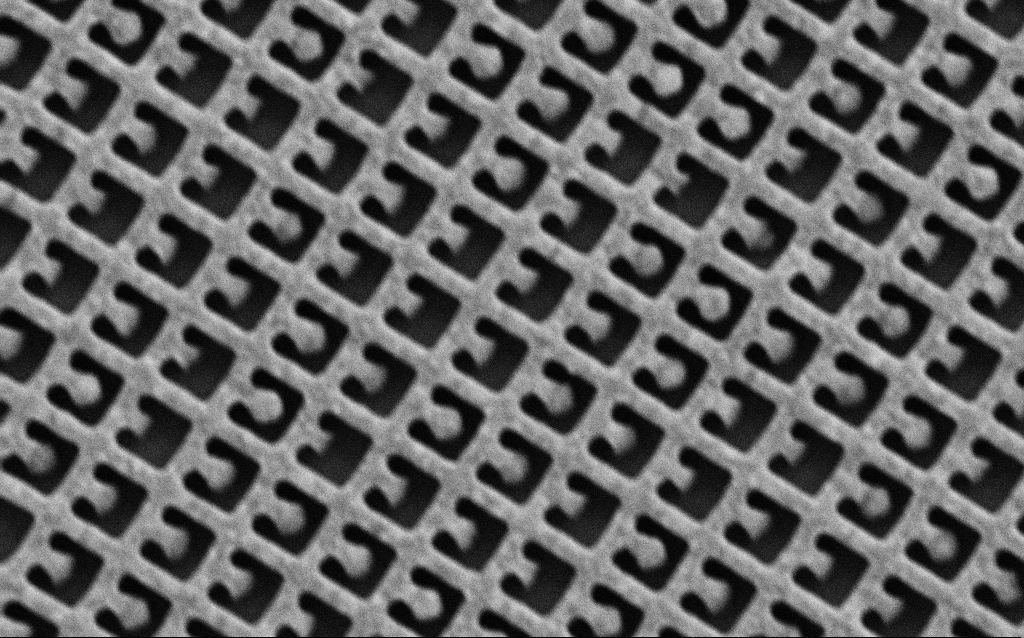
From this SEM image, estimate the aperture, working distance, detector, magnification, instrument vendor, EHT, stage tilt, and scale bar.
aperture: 30 µm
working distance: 6.1 mm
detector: SE2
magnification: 64.72 K X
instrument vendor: Zeiss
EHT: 5 kV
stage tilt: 0°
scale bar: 1000 nm